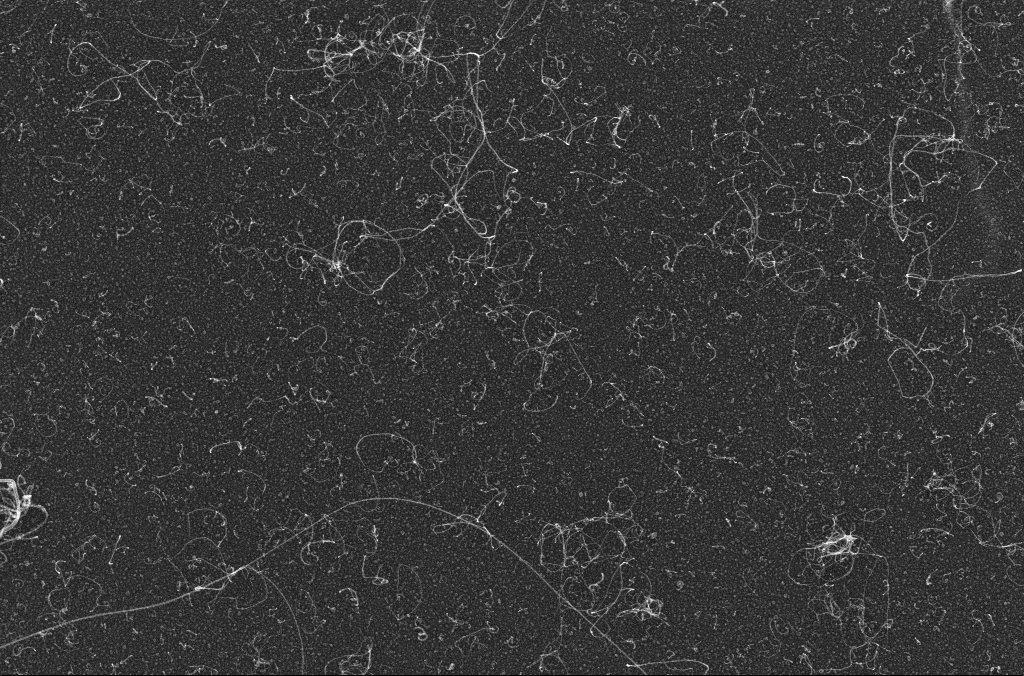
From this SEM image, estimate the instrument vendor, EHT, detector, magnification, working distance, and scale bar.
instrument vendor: Zeiss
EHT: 10 kV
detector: InLens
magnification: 50 K X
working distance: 3.2 mm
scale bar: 1000 nm